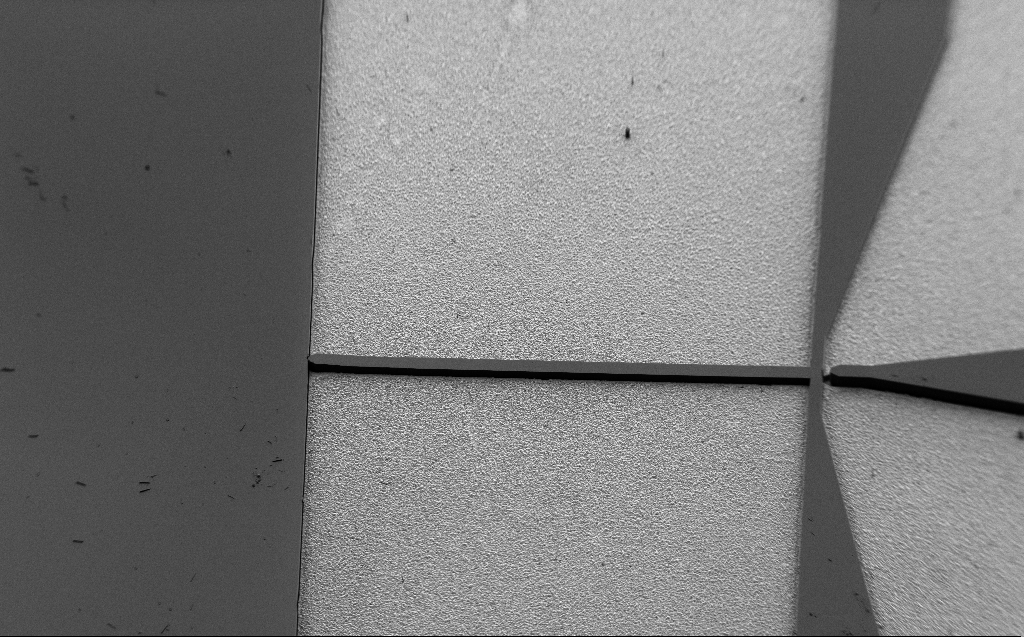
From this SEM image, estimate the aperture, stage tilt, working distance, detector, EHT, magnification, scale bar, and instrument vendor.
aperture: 30 µm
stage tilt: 45°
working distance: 7 mm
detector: SE2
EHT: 1 kV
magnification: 0.36 K X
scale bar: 200000 nm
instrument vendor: Zeiss